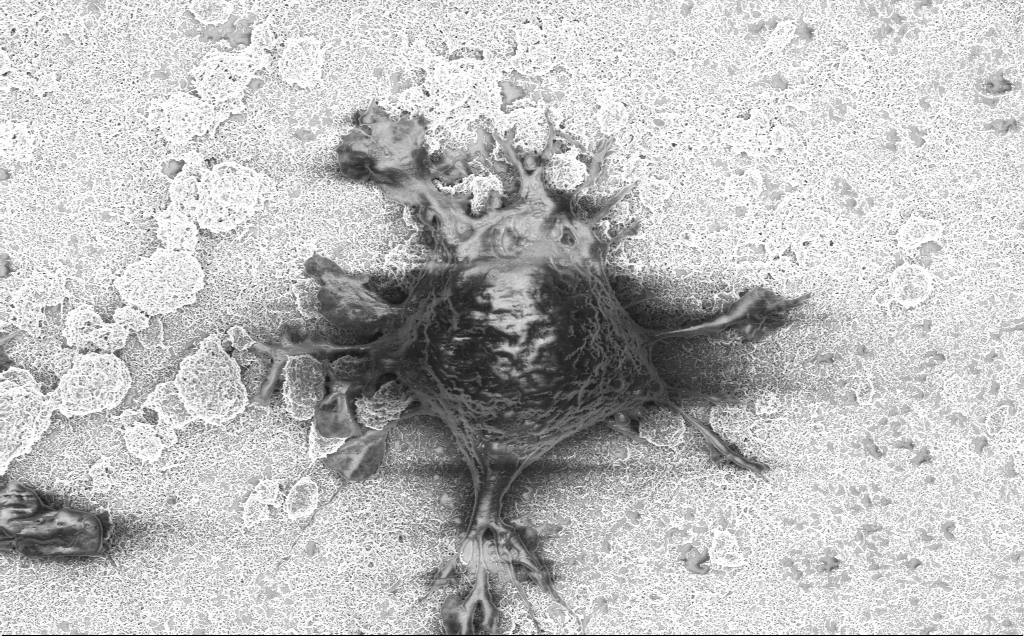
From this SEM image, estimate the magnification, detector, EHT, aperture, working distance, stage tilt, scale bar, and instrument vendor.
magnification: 7 K X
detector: InLens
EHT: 2 kV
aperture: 30 µm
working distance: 7.1 mm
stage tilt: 0°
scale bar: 10000 nm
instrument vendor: Zeiss